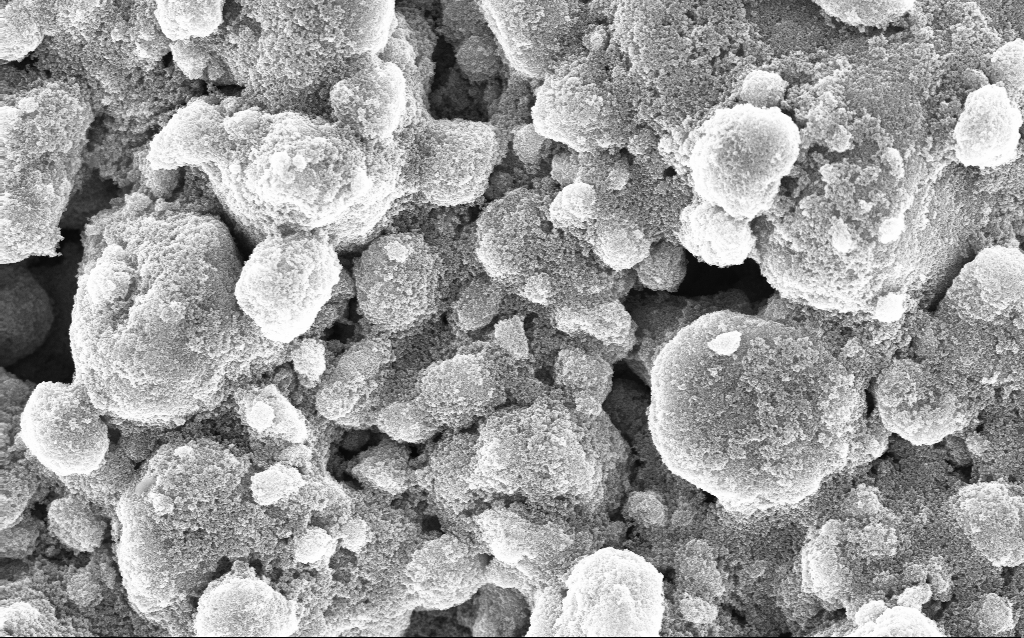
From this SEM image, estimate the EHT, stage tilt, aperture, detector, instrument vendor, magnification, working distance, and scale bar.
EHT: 10 kV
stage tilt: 0°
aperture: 30 µm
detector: InLens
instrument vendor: Zeiss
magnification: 16 K X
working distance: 3.8 mm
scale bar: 2000 nm